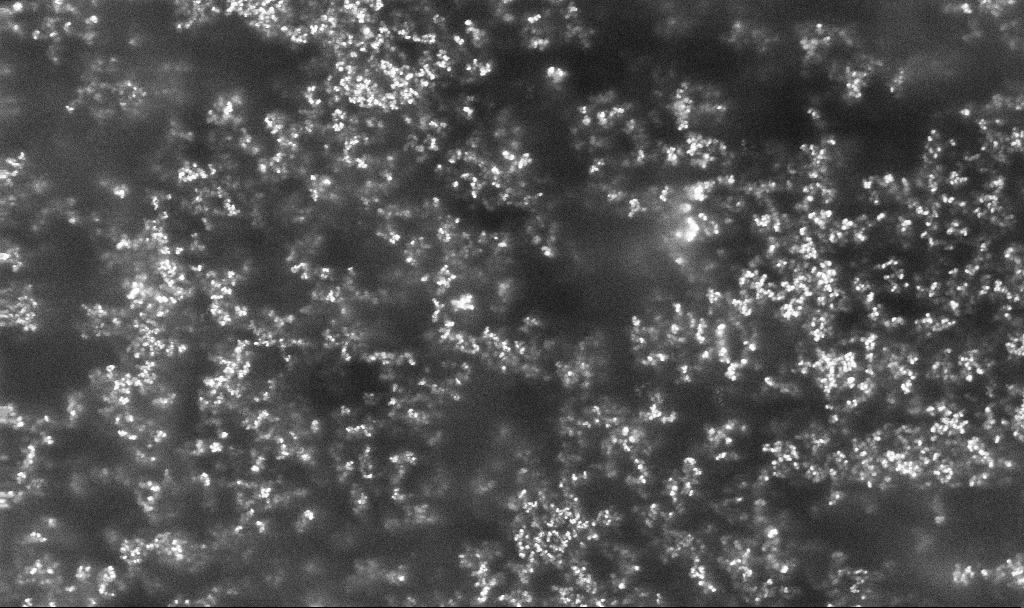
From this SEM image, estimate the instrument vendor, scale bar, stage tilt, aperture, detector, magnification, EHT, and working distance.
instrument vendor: Zeiss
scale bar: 1000 nm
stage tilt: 0°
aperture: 30 µm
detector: InLens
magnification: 16.55 K X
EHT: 10 kV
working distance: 2.4 mm